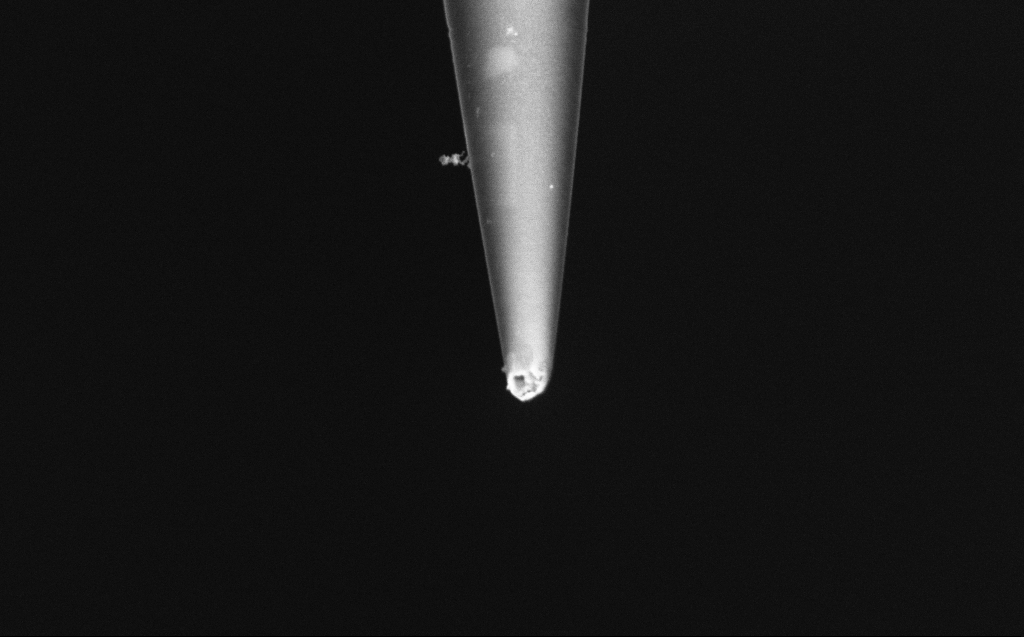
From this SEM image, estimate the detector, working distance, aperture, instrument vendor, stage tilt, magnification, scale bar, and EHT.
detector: InLens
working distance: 6 mm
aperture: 30 µm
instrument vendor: Zeiss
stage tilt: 45°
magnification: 50 K X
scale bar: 1000 nm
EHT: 2 kV